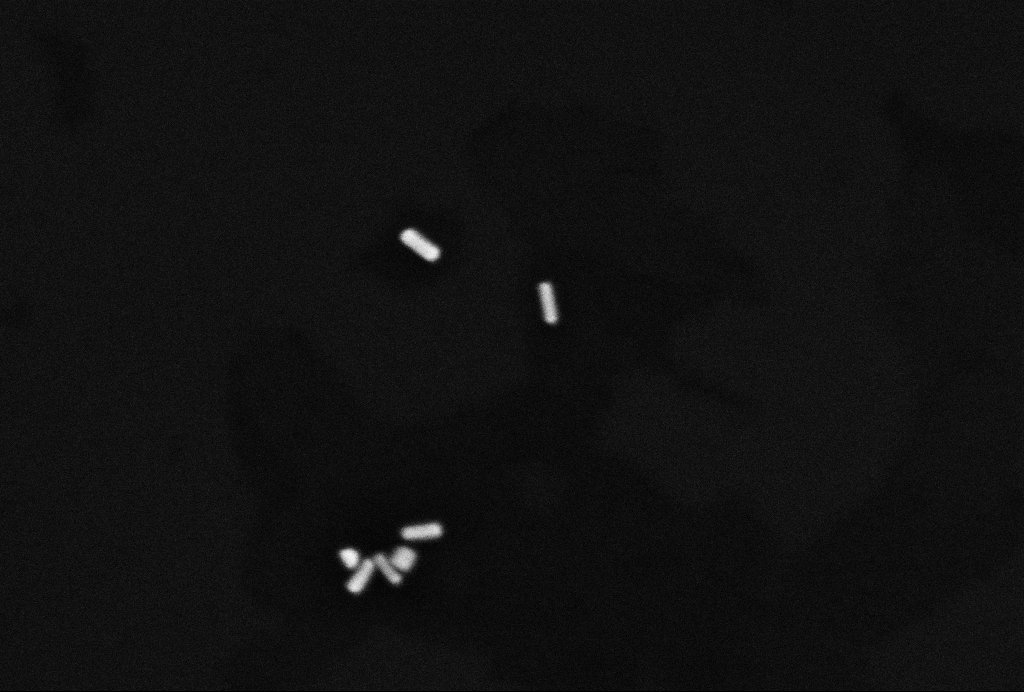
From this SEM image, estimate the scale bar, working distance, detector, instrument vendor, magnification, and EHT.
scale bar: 200 nm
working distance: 3.2 mm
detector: InLens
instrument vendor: Zeiss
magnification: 200 K X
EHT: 10 kV